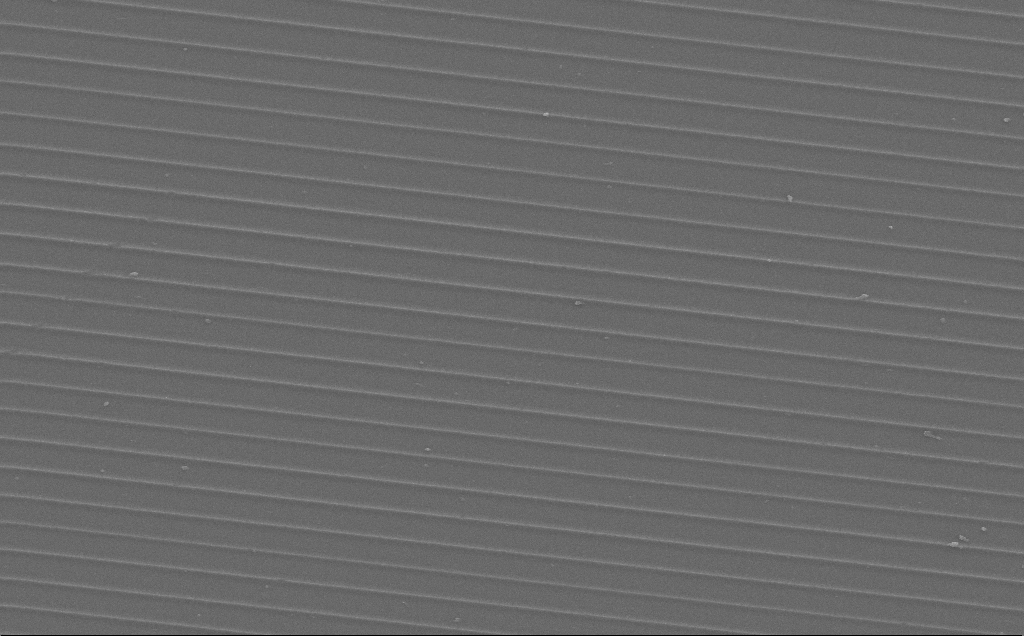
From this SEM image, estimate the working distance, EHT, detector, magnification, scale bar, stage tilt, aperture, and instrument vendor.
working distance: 11 mm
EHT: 10 kV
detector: SE2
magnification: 1.6 K X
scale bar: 10000 nm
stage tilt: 0°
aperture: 30 µm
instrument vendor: Zeiss